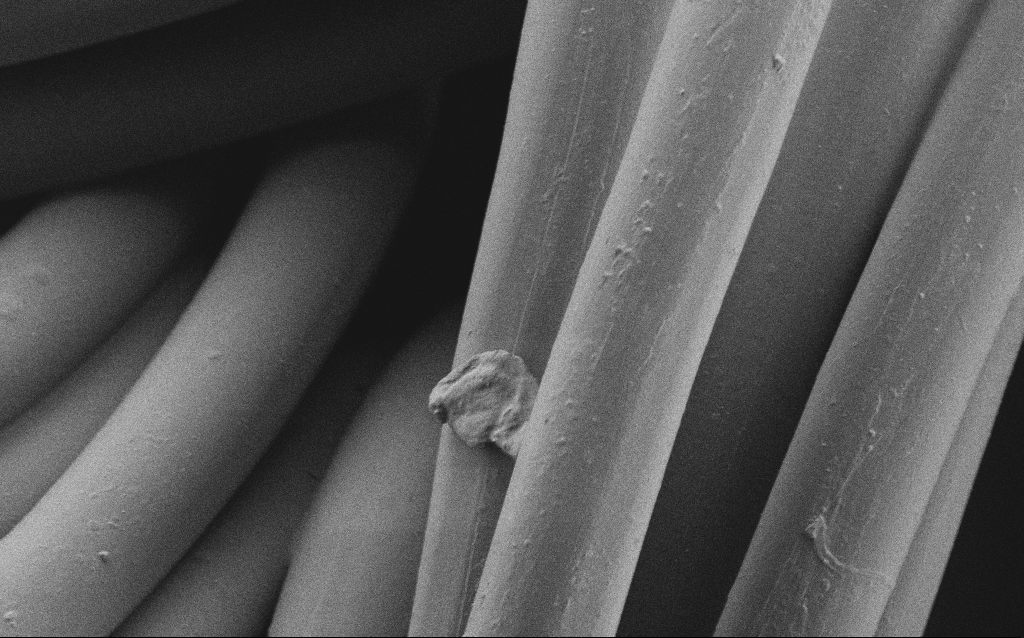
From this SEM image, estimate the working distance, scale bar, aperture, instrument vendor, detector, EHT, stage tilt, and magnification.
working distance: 4 mm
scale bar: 20000 nm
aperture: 30 µm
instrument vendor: Zeiss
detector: SE2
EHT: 1 kV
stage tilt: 0°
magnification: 2.33 K X